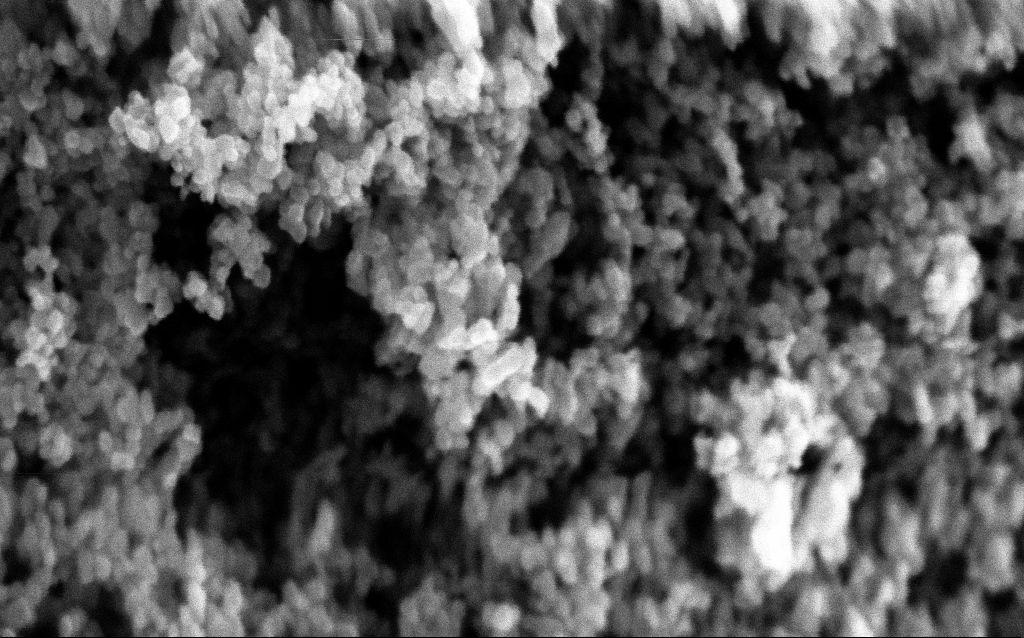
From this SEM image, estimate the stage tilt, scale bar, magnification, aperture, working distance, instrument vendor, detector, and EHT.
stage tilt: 0°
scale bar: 100 nm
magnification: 204.13 K X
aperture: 30 µm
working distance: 2.5 mm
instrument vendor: Zeiss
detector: InLens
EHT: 5 kV